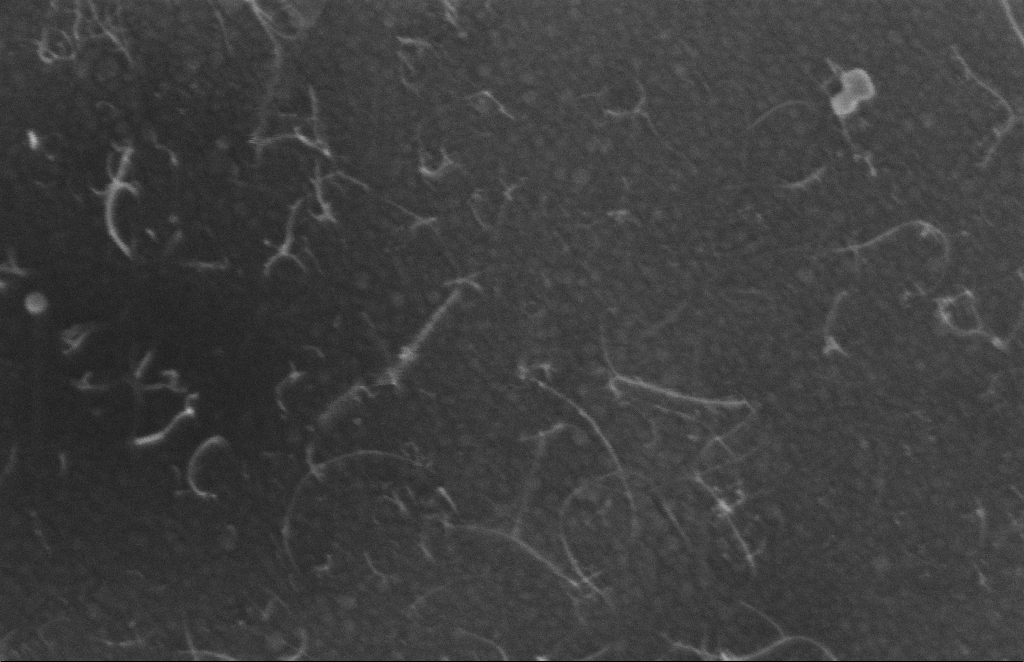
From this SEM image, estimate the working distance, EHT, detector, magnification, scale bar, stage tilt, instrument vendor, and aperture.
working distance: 8 mm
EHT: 5 kV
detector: InLens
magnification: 270.4 K X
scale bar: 100 nm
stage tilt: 0°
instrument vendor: Zeiss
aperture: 20 µm